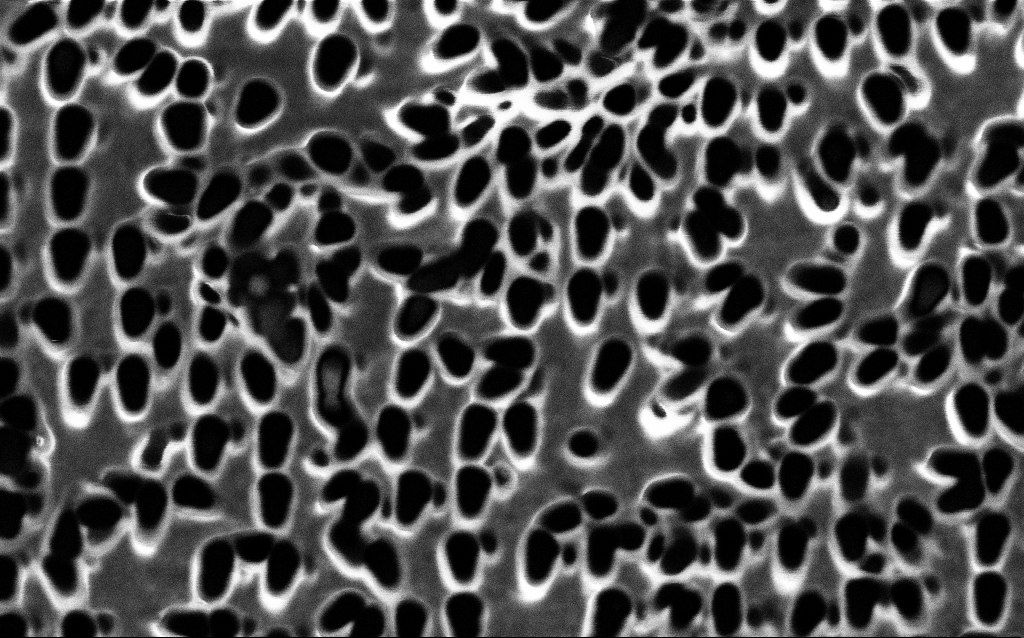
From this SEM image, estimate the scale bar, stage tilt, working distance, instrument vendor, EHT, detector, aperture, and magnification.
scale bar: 1000 nm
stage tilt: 0°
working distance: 8 mm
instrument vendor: Zeiss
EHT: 1.5 kV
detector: SE2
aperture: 30 µm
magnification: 51.06 K X